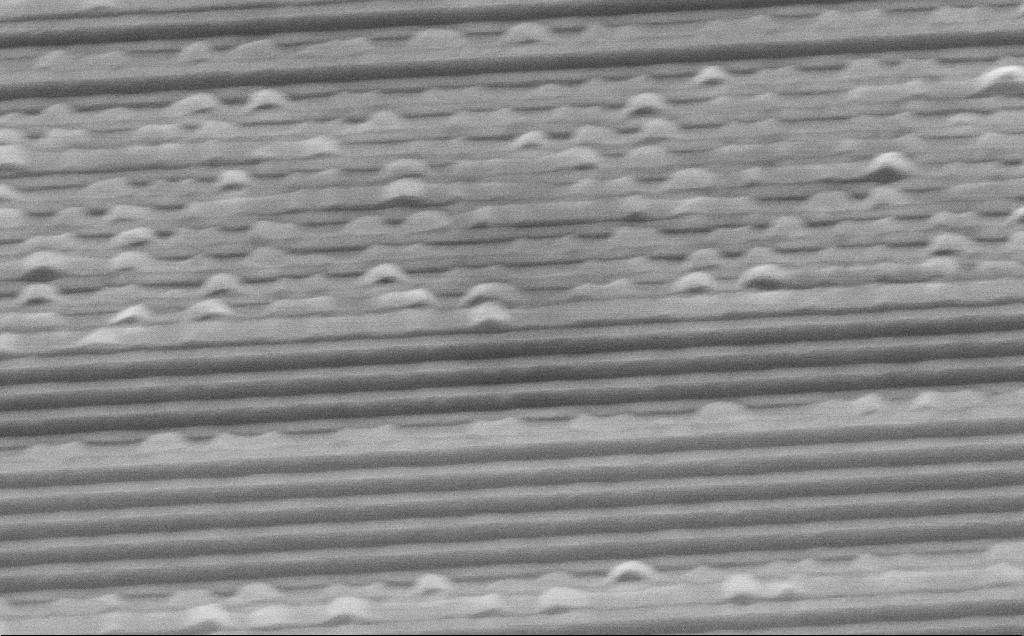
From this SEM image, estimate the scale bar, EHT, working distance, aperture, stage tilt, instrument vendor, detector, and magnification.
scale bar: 1000 nm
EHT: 10 kV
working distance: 7 mm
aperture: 30 µm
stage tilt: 45°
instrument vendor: Zeiss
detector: InLens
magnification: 53.66 K X